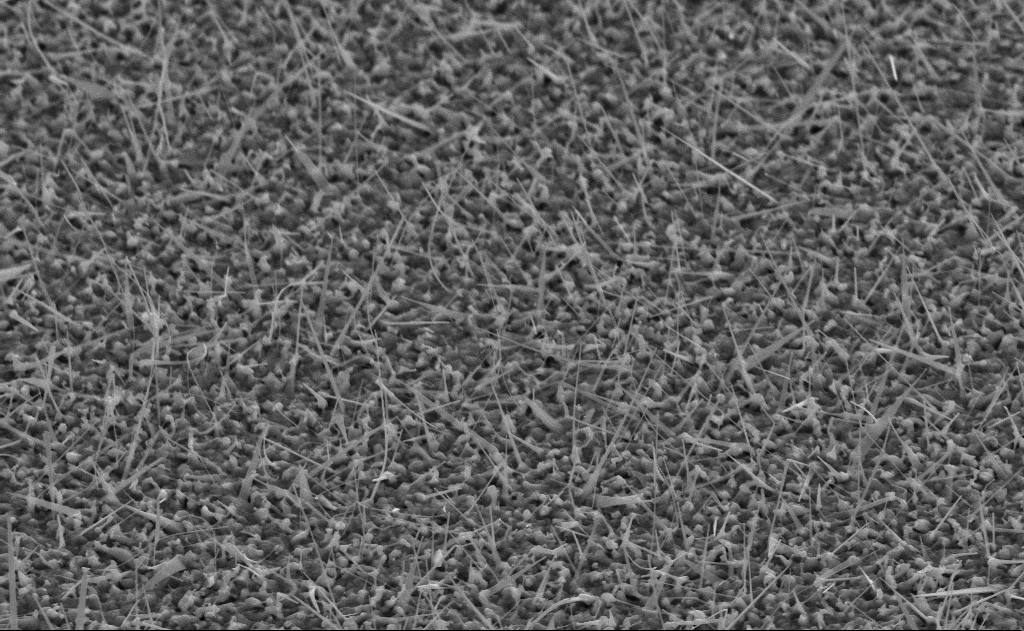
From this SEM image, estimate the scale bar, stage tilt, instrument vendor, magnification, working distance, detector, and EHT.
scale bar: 1000 nm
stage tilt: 45°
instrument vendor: Zeiss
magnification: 20 K X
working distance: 11 mm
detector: SE2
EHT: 10 kV